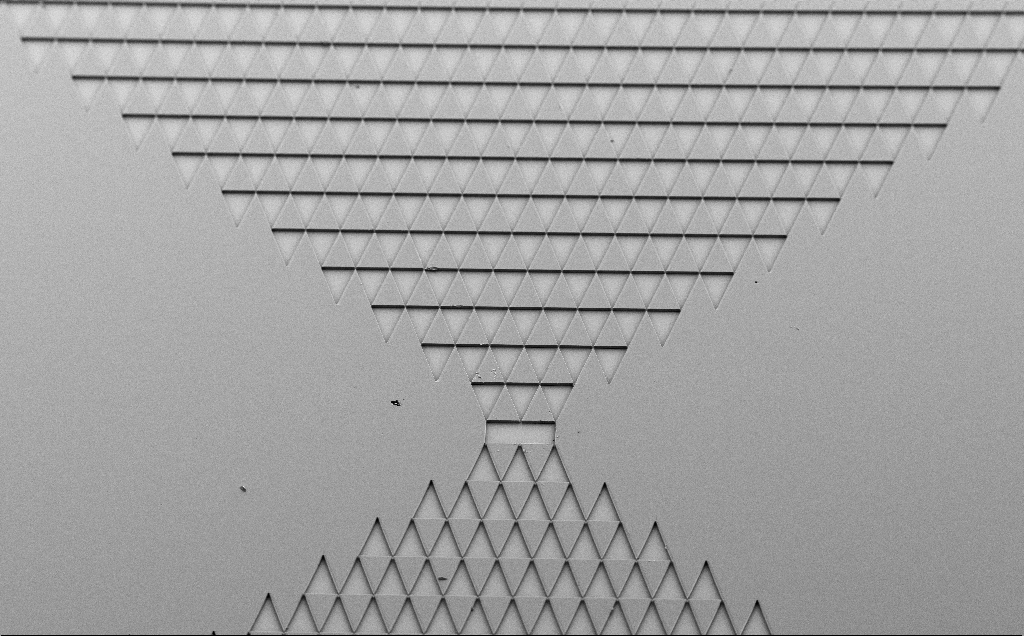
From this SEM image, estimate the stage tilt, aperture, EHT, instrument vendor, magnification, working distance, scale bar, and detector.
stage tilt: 40°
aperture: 30 µm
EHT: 5 kV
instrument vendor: Zeiss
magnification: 0.212 K X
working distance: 9 mm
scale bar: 100000 nm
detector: SE2